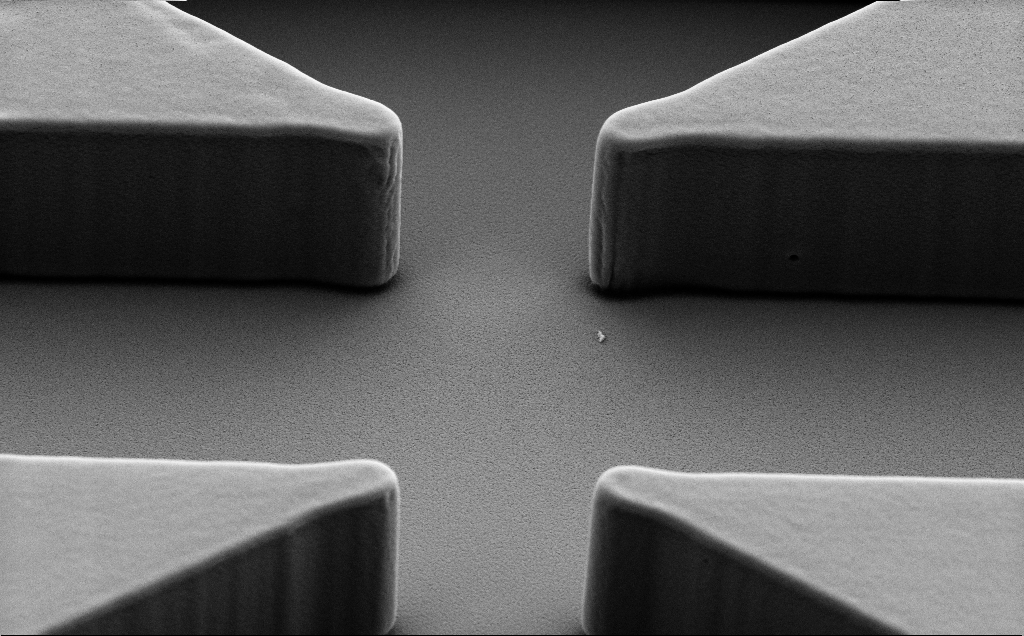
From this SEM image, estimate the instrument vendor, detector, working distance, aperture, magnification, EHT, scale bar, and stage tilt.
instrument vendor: Zeiss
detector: SE2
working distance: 9 mm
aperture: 30 µm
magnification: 12.12 K X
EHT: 5 kV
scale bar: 1000 nm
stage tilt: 40°